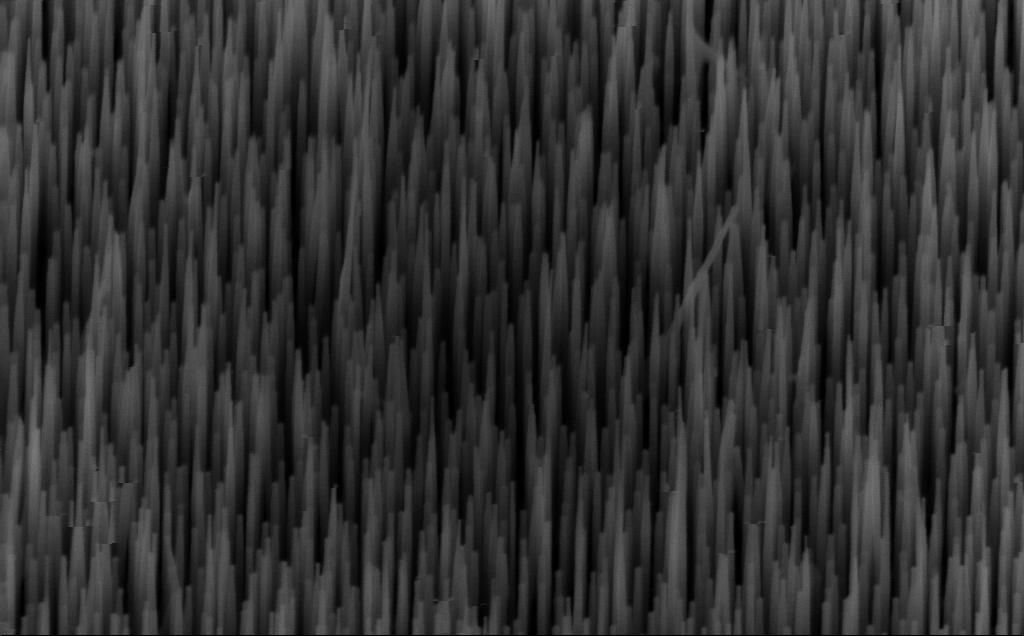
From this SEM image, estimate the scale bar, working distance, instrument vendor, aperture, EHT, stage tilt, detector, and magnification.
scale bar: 200 nm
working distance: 5 mm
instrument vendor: Zeiss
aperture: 30 µm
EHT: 10 kV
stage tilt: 45°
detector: InLens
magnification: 80 K X